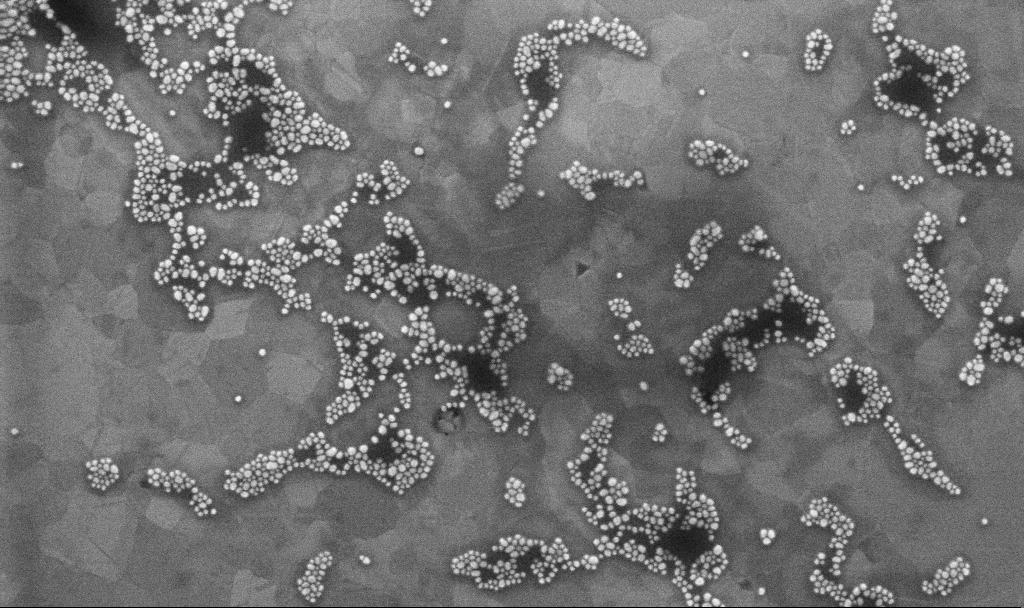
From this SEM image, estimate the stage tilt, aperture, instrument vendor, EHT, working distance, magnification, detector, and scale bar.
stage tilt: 0°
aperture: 30 µm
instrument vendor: Zeiss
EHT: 10 kV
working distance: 3.1 mm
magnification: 110.18 K X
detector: InLens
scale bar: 200 nm